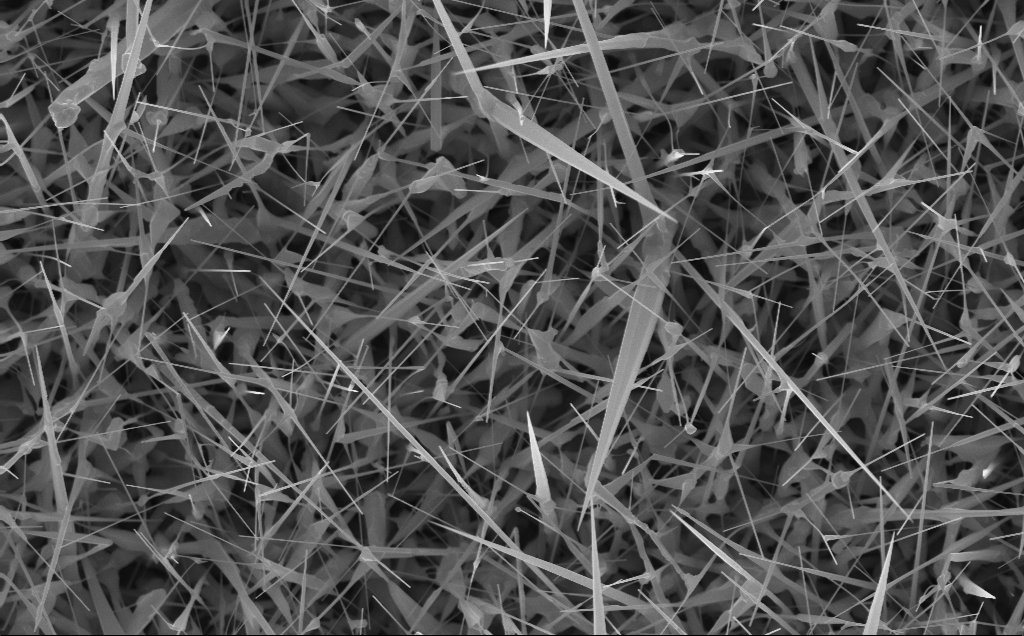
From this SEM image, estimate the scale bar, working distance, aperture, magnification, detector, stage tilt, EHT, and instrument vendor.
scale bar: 2000 nm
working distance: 4 mm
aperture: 30 µm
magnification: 20 K X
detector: InLens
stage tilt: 0°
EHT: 10 kV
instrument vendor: Zeiss